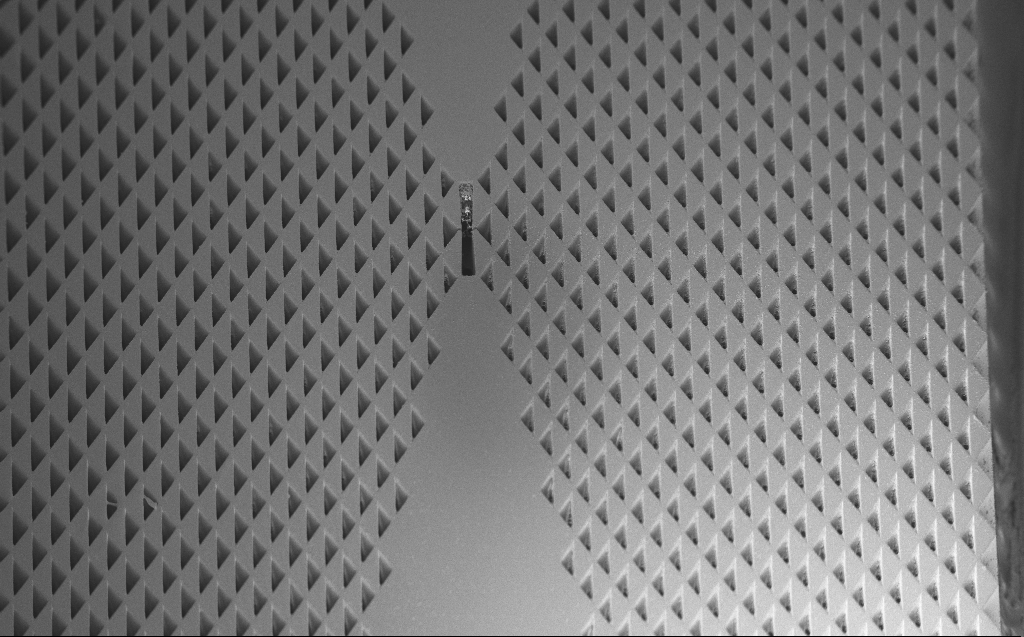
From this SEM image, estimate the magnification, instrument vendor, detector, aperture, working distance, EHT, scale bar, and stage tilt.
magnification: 0.188 K X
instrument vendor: Zeiss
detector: InLens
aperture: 30 µm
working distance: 7 mm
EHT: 3 kV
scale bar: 100000 nm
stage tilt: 45°